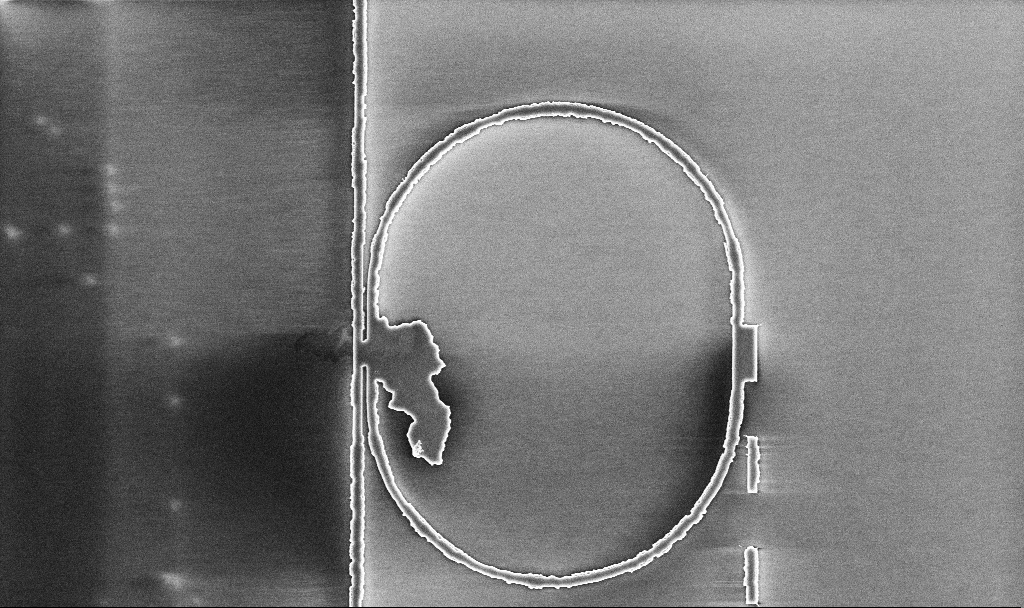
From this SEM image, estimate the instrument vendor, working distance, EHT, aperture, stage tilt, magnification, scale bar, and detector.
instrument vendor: Zeiss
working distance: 10.1 mm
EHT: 5 kV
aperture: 30 µm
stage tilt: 0°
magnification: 6.94 K X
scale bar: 10000 nm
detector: InLens